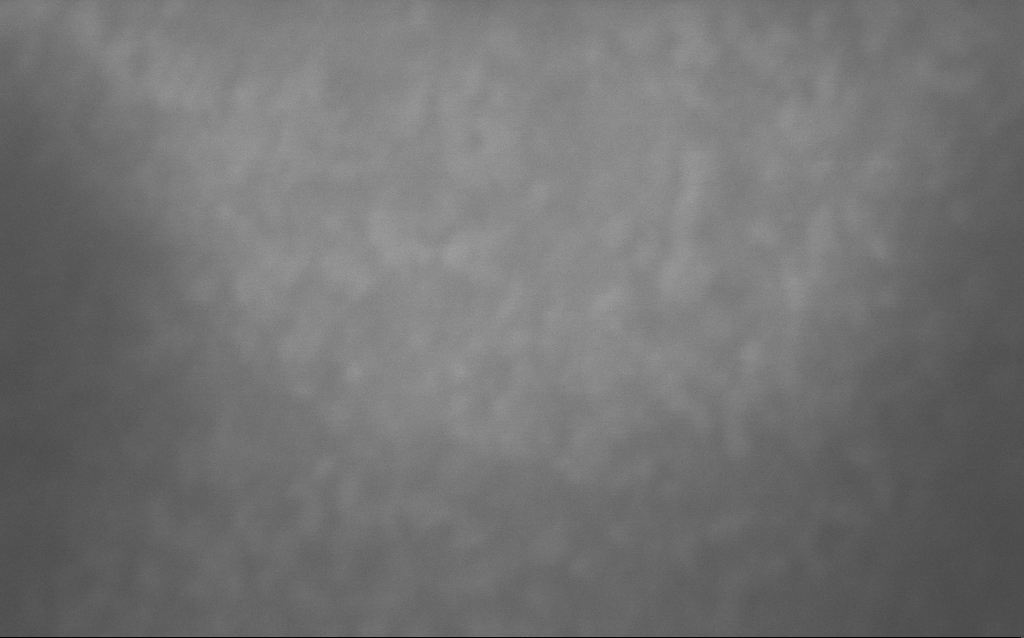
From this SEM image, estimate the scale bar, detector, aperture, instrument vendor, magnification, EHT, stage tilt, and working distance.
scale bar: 20 nm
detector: InLens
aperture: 30 µm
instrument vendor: Zeiss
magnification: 1585.7 K X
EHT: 10 kV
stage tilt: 0°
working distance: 3 mm